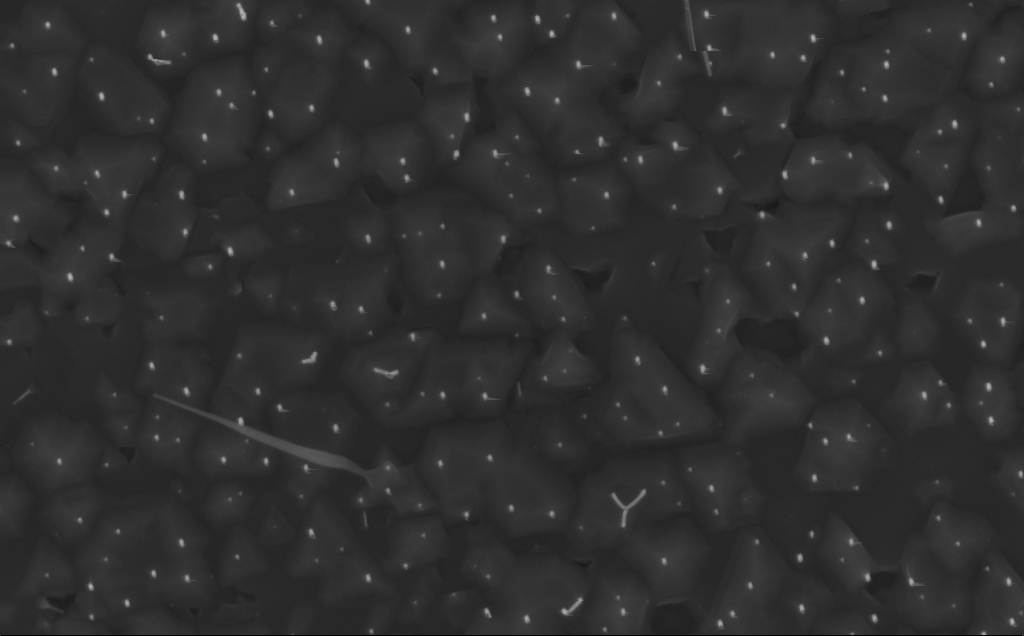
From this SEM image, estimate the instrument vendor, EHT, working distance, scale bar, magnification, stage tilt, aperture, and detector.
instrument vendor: Zeiss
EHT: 10 kV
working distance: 3 mm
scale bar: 1000 nm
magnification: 40 K X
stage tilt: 0°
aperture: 30 µm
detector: InLens